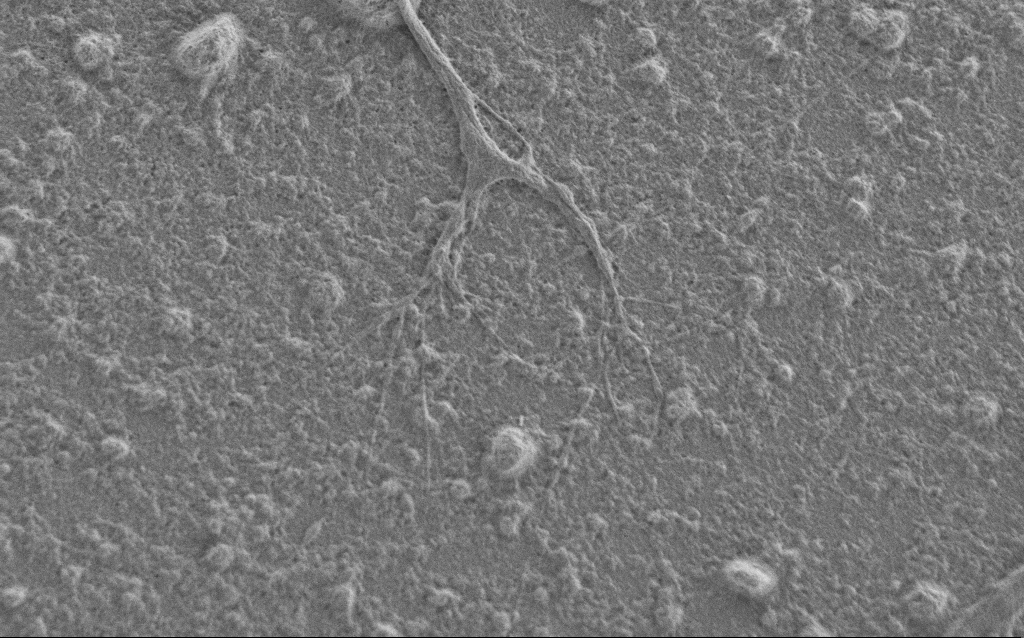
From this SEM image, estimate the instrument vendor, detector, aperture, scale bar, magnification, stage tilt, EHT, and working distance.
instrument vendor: Zeiss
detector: SE2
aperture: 30 µm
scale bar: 2000 nm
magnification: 7.5 K X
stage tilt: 0°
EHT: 1 kV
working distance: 6 mm